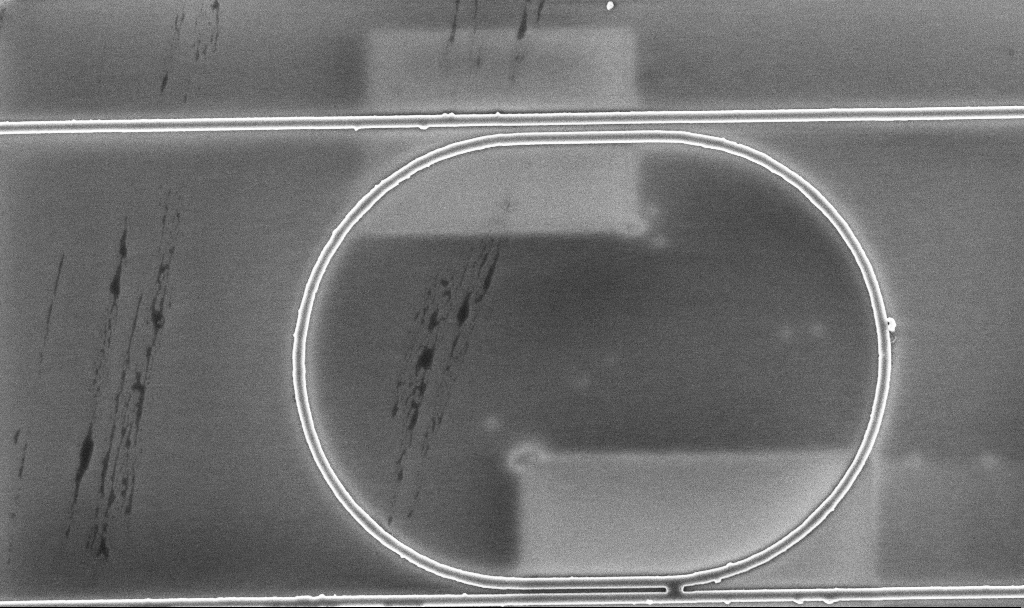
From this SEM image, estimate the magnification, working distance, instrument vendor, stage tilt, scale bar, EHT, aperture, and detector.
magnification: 8.54 K X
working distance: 5.2 mm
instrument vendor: Zeiss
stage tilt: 0°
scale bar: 2000 nm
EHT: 5 kV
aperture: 30 µm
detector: InLens